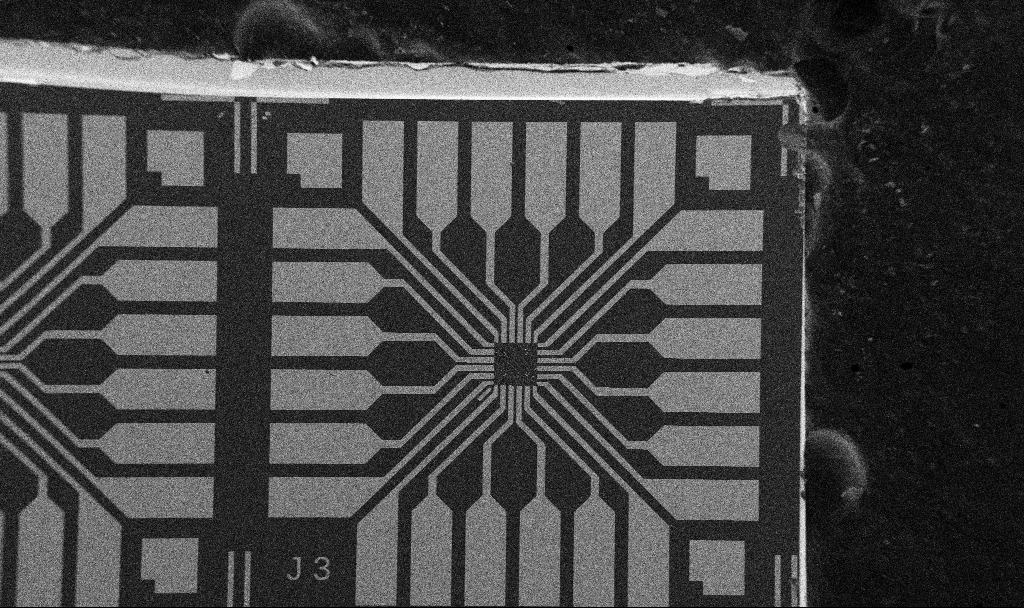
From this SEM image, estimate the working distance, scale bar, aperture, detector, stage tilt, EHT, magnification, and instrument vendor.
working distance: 10.7 mm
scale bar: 200000 nm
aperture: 30 µm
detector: SE2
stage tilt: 0°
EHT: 5 kV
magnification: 0.1 K X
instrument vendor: Zeiss